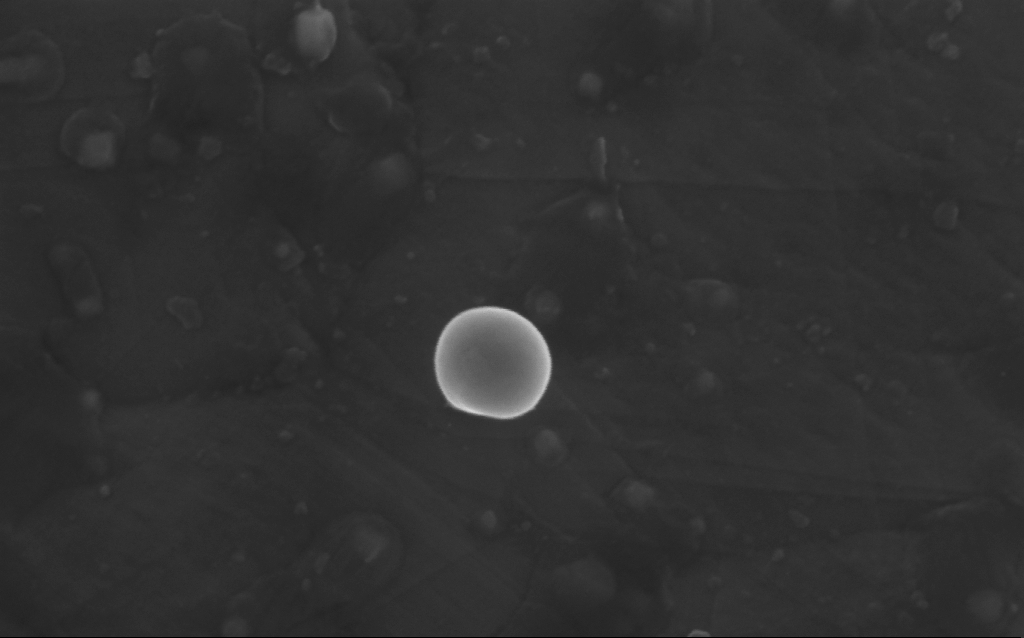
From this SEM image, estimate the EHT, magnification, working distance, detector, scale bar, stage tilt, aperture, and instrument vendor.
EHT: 5 kV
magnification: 200.75 K X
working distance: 4 mm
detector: InLens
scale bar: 200 nm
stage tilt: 0°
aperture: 30 µm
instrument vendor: Zeiss